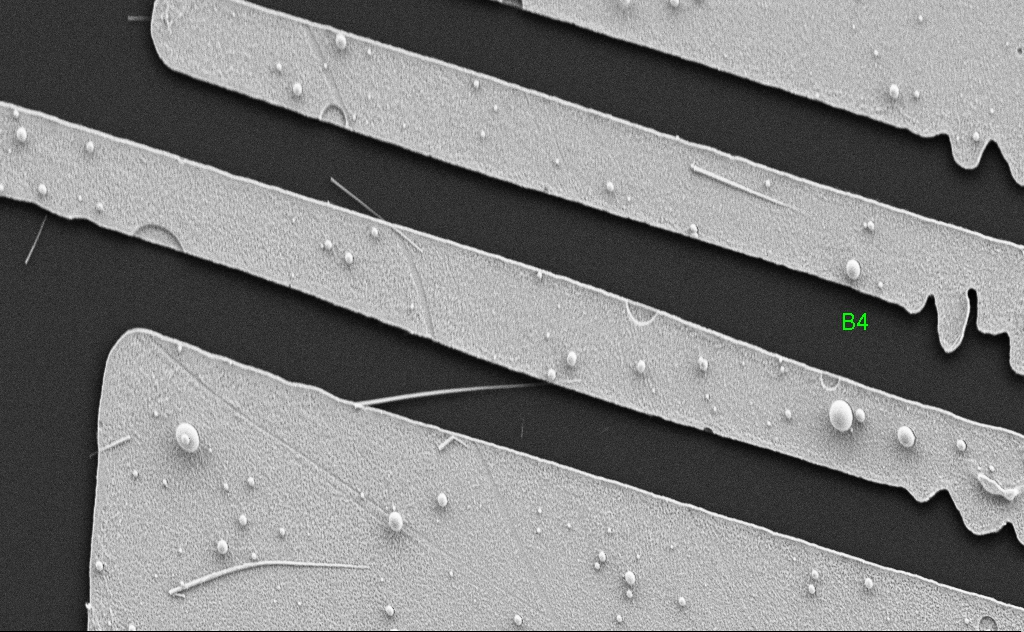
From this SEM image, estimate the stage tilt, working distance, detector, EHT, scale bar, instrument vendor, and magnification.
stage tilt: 0°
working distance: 5 mm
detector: SE2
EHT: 5 kV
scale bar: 2000 nm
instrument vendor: Zeiss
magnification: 12.5 K X